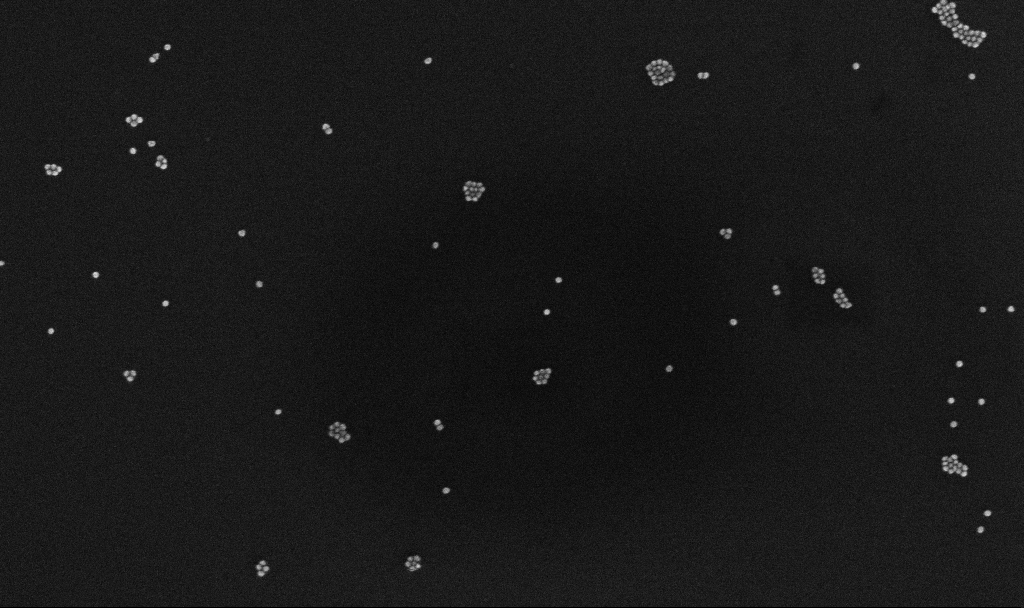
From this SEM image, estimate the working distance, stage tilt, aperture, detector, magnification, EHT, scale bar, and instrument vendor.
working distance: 3.3 mm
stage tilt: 0°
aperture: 30 µm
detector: InLens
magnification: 100 K X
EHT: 10 kV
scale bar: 200 nm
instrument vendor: Zeiss